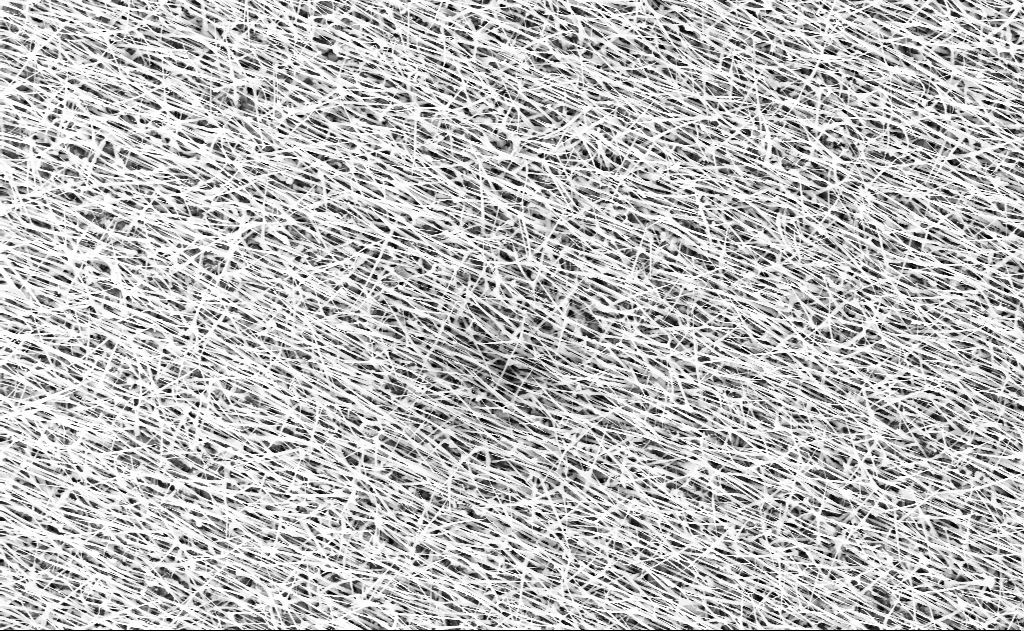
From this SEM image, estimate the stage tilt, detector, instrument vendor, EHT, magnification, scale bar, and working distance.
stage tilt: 0°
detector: InLens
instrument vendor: Zeiss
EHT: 10 kV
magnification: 10 K X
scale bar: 2000 nm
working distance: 13 mm